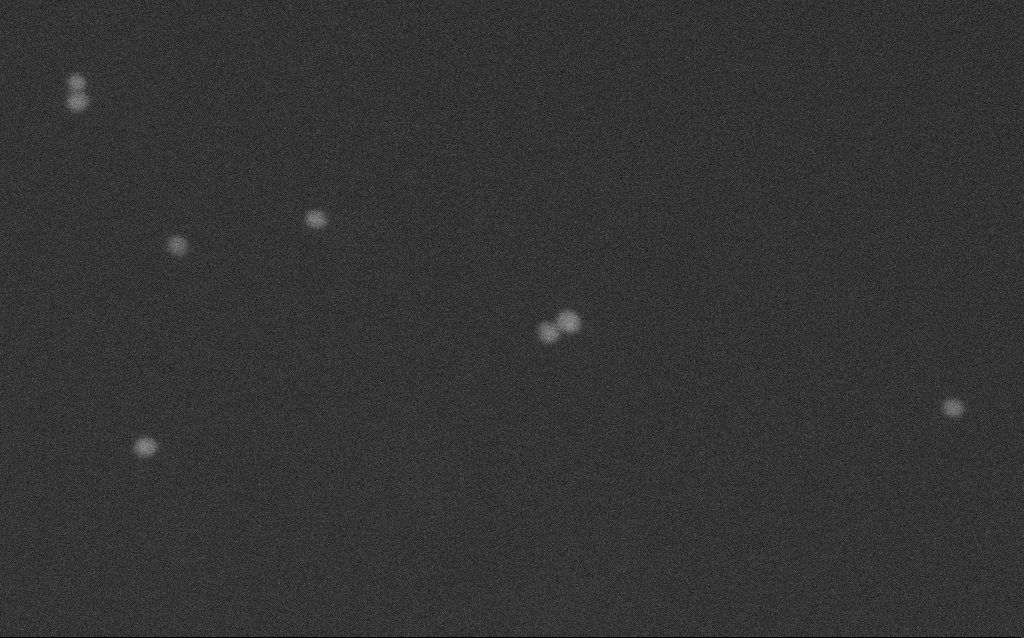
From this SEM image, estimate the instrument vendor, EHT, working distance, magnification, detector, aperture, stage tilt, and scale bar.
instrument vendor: Zeiss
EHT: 8 kV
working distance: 2.7 mm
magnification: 673.76 K X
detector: SE2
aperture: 30 µm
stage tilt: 0°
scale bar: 100 nm